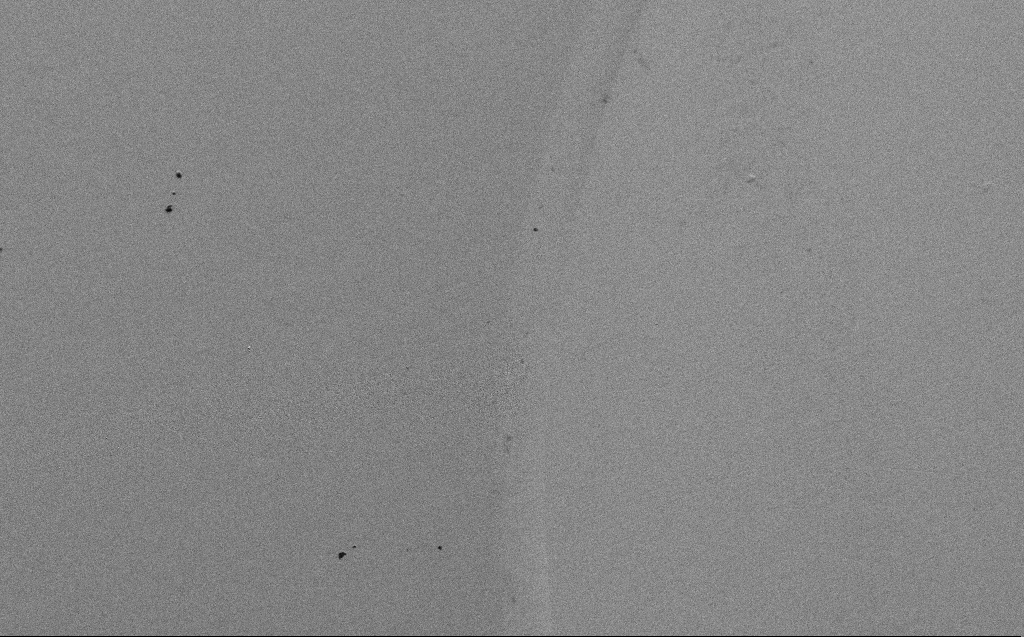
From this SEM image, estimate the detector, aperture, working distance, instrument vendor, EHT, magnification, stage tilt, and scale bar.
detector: SE2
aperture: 30 µm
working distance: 8 mm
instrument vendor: Zeiss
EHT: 5 kV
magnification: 0.45 K X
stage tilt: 45°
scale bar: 100000 nm